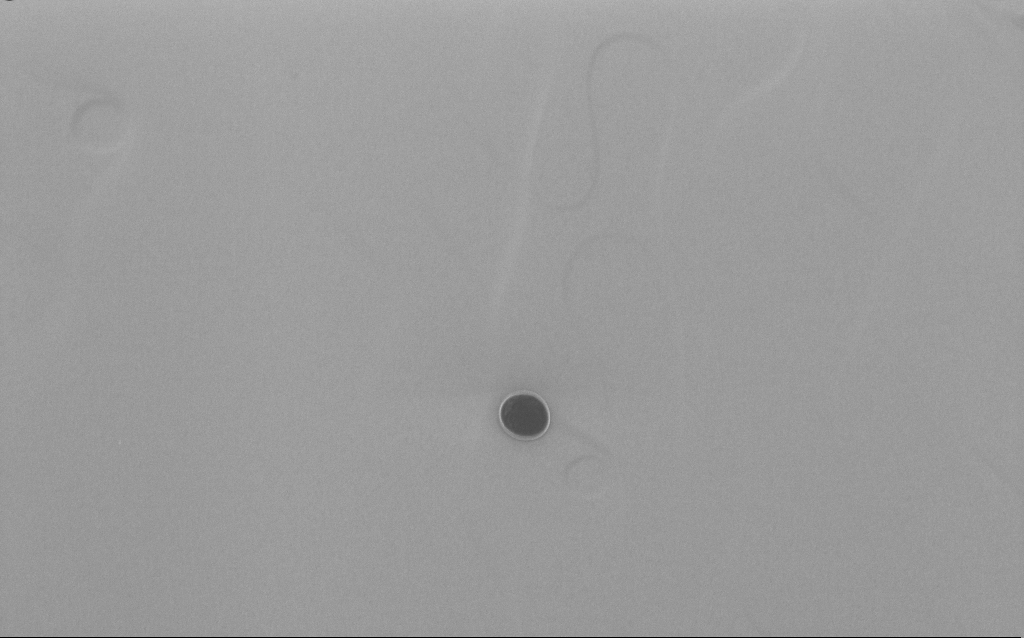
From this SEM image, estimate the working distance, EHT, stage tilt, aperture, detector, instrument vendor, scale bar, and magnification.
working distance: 4 mm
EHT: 5 kV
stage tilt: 20°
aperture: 30 µm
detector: InLens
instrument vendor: Zeiss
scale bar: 2000 nm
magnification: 17.24 K X